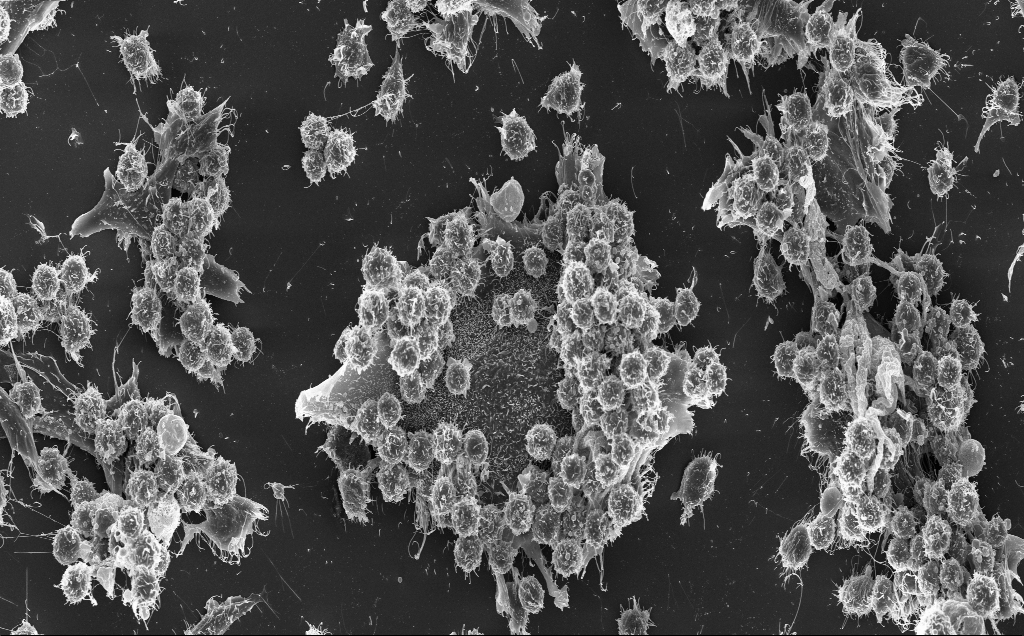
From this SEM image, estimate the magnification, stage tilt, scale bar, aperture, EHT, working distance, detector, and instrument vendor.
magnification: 1.3 K X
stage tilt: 0.7°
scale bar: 20000 nm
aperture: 30 µm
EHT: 10 kV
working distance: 5 mm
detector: InLens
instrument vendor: Zeiss